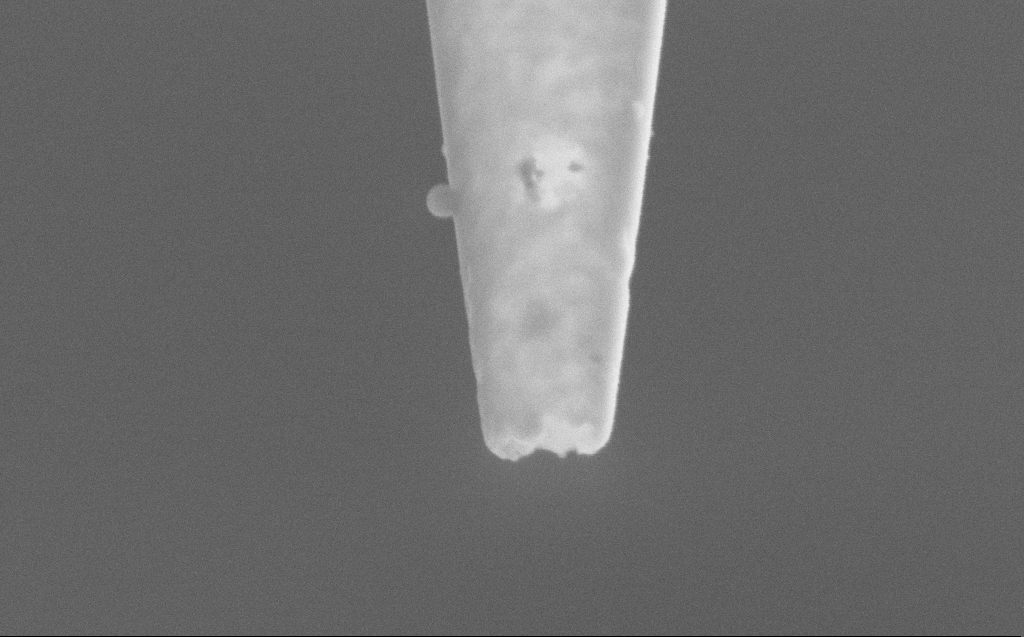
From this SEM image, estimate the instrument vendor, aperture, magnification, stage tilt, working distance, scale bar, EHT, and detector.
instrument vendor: Zeiss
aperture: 30 µm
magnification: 106.97 K X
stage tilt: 45°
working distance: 4 mm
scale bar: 200 nm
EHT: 5 kV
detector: SE2